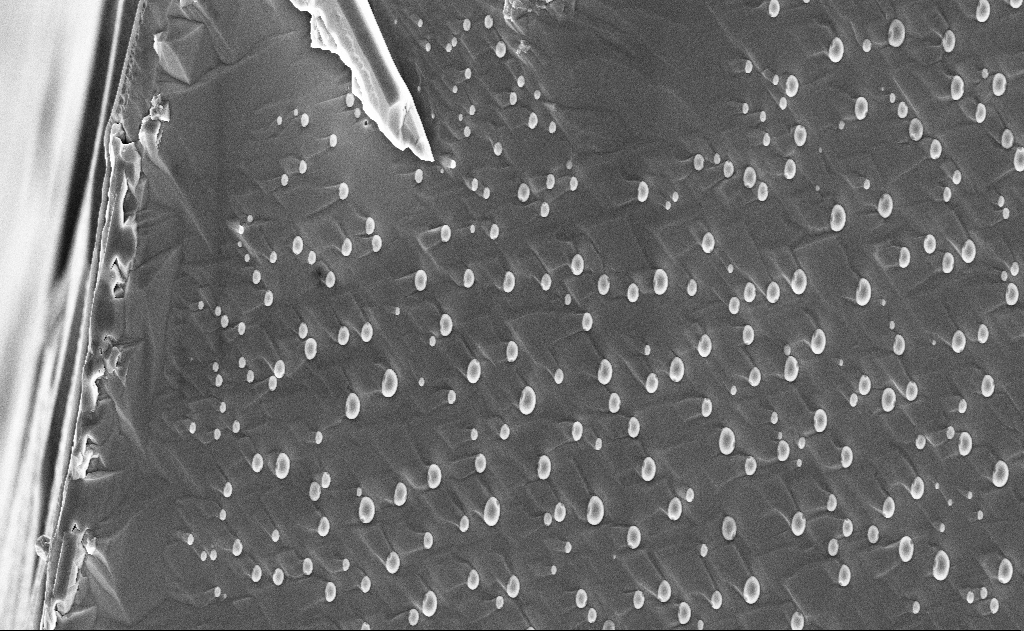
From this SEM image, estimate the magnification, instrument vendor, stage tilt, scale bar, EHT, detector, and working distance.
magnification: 5 K X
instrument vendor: Zeiss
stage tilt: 0°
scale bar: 10000 nm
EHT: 10 kV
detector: InLens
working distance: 15 mm